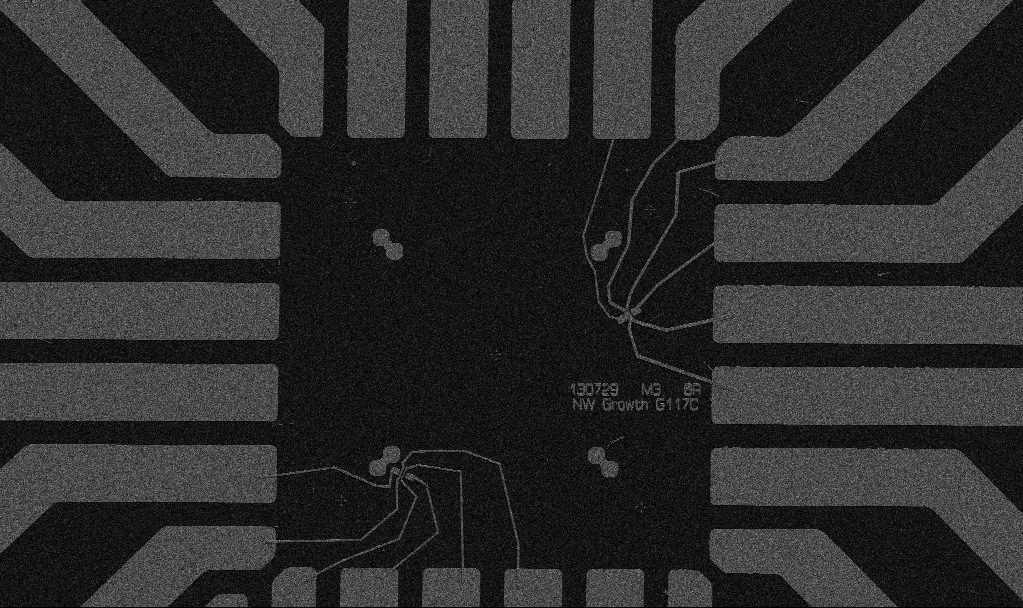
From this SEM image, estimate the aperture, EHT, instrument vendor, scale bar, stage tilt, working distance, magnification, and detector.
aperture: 30 µm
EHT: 5 kV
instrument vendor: Zeiss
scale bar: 20000 nm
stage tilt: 0°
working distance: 10.7 mm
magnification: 1 K X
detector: SE2